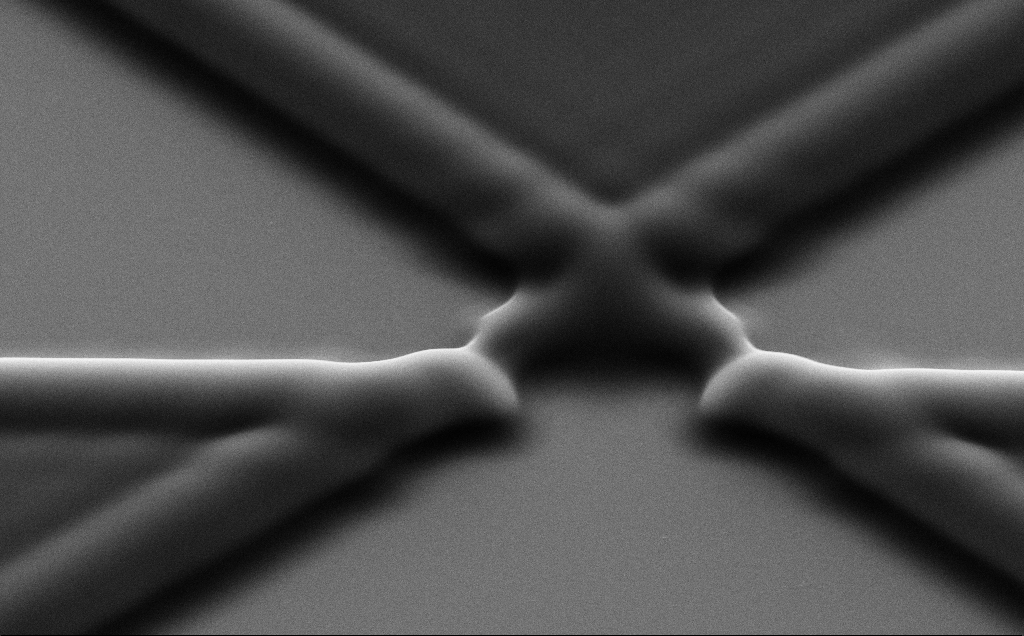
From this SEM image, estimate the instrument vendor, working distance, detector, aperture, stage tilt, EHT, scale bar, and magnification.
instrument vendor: Zeiss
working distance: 6 mm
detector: SE2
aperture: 30 µm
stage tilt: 35°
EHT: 7 kV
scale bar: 2000 nm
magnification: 13.97 K X